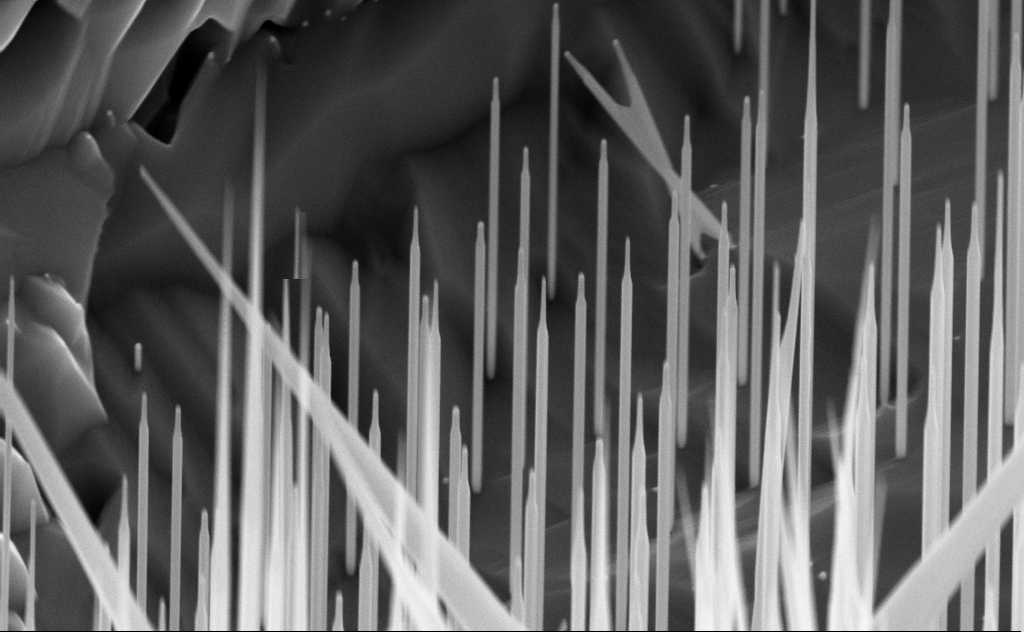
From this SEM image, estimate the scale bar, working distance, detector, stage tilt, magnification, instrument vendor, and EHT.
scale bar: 1000 nm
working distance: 6 mm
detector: InLens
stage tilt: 45°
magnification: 51.87 K X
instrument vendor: Zeiss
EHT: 10 kV